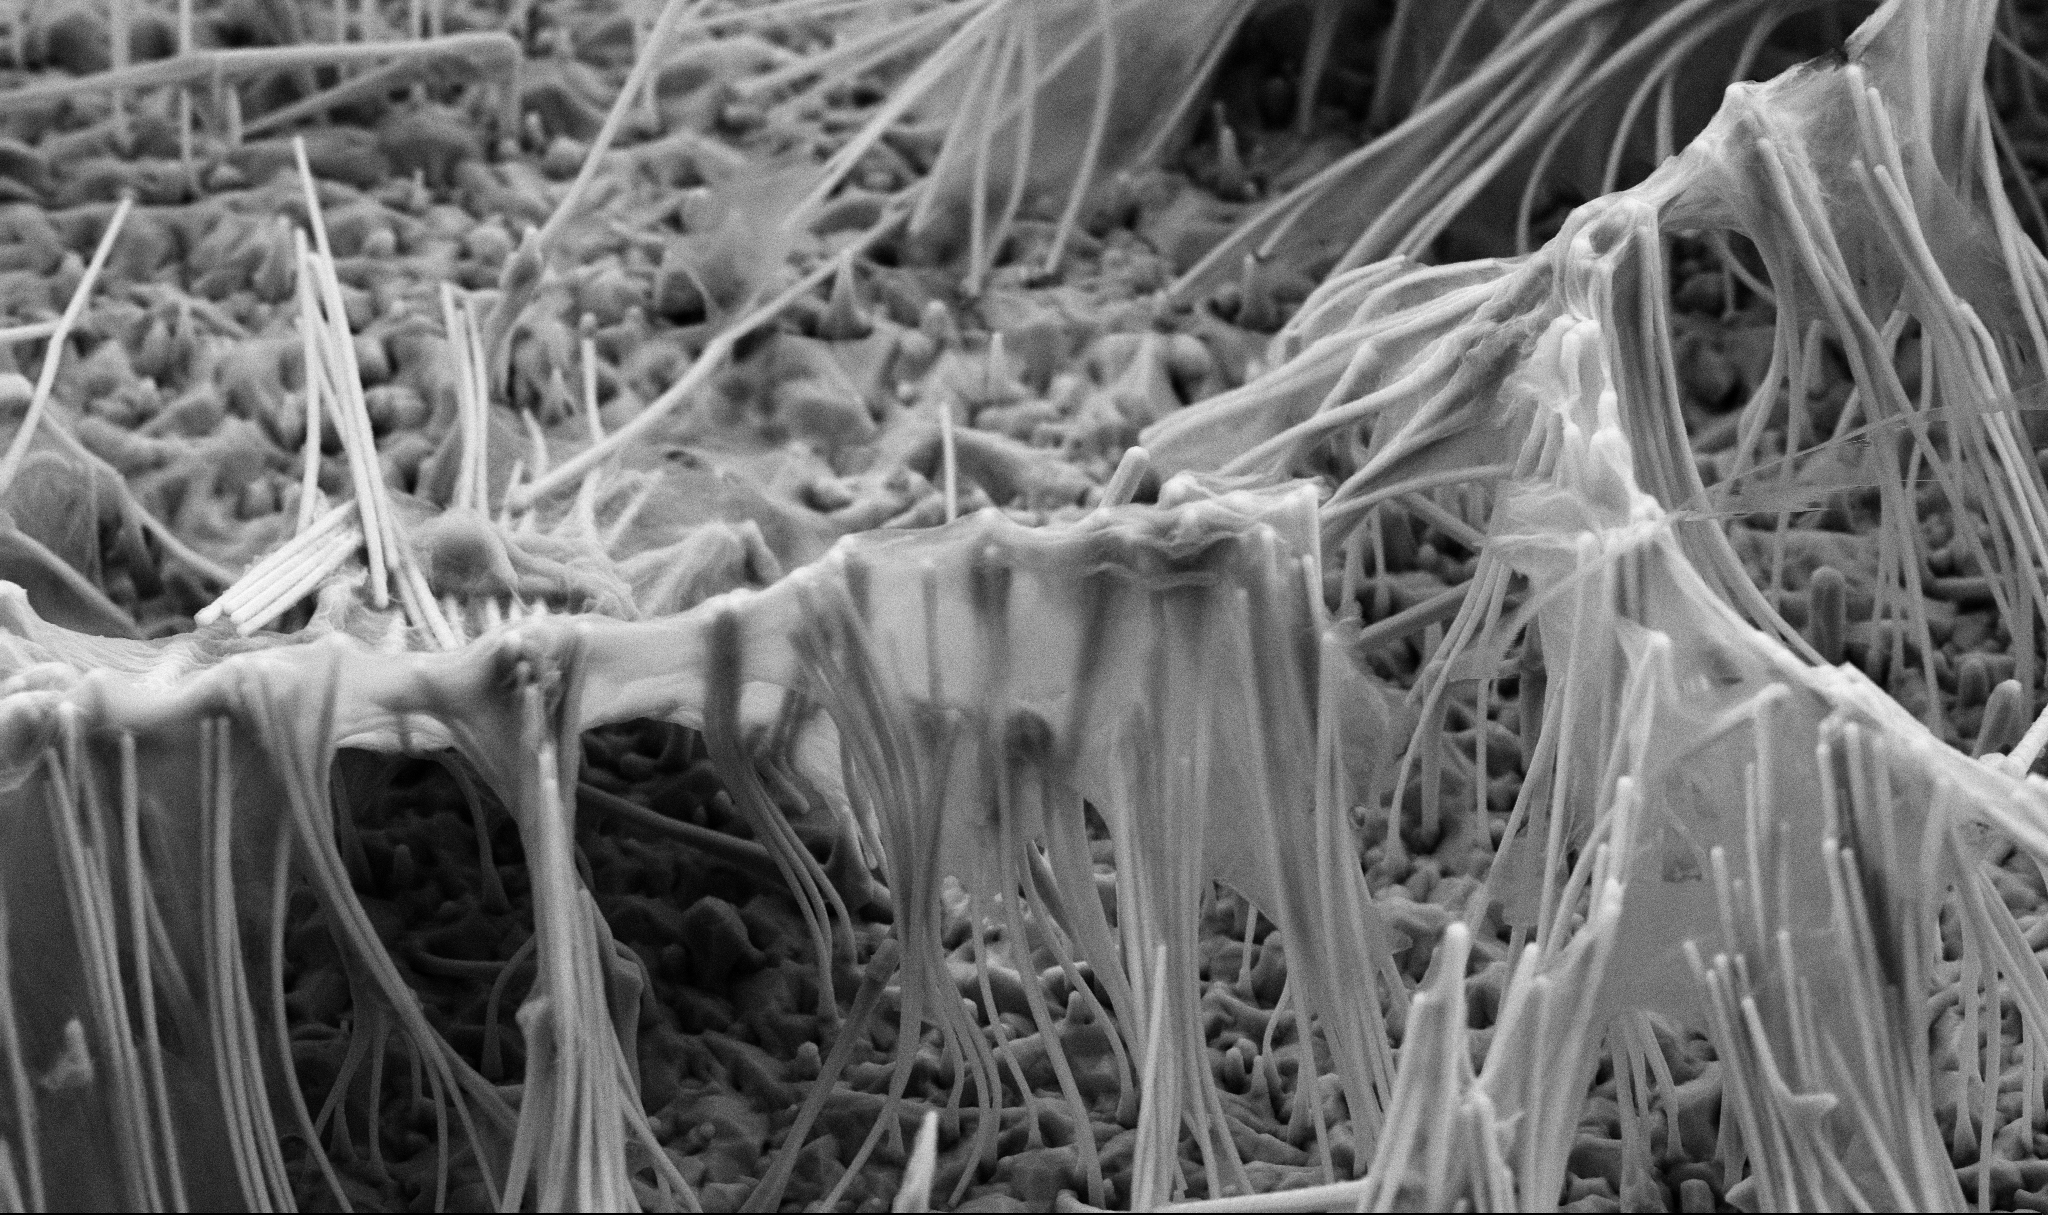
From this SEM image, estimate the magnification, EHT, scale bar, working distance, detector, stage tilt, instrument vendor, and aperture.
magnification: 20 K X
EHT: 5 kV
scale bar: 2000 nm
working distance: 7.2 mm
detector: SE2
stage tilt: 45°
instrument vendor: Zeiss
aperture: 30 µm